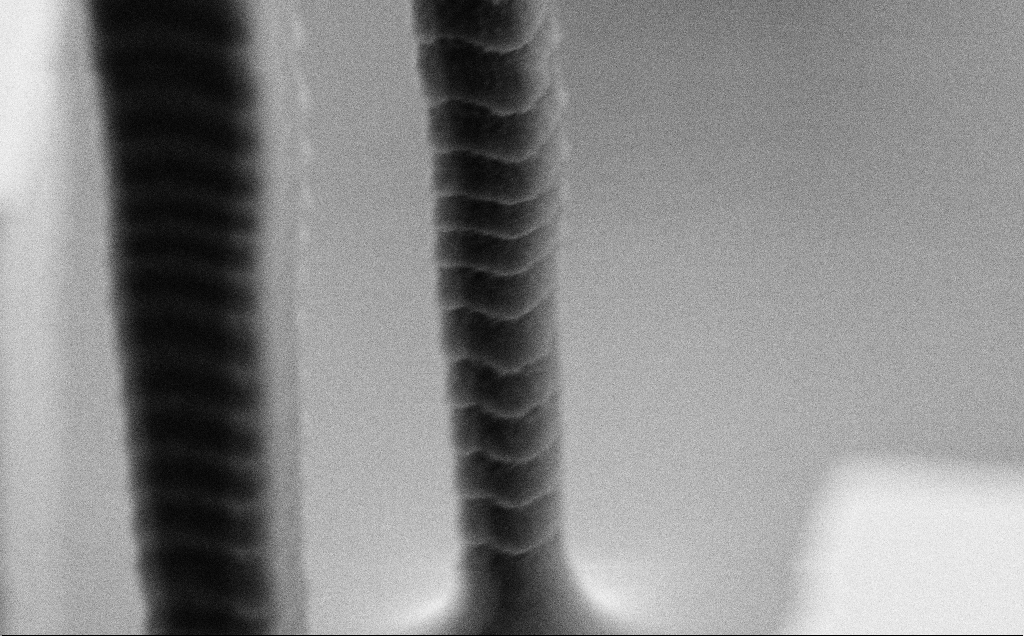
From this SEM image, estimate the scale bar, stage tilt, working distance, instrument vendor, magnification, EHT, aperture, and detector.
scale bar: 1000 nm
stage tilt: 60°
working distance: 5.3 mm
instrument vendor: Zeiss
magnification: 47.28 K X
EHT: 3 kV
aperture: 30 µm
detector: SE2